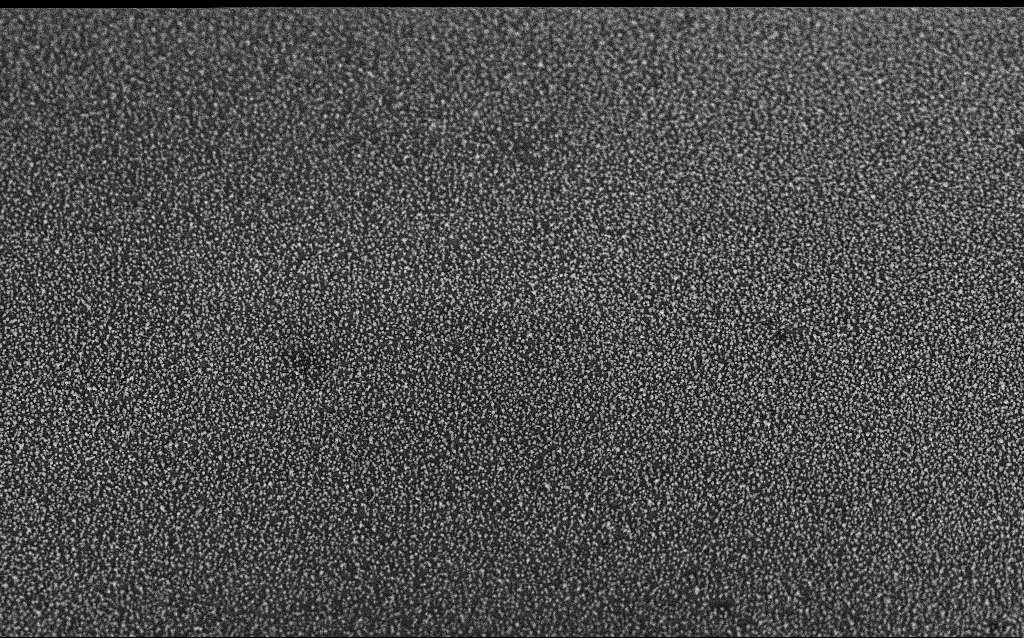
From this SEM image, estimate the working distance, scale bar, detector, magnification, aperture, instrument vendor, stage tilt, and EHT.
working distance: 6.2 mm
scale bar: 2000 nm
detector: InLens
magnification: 10 K X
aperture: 30 µm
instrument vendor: Zeiss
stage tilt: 45°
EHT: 5 kV